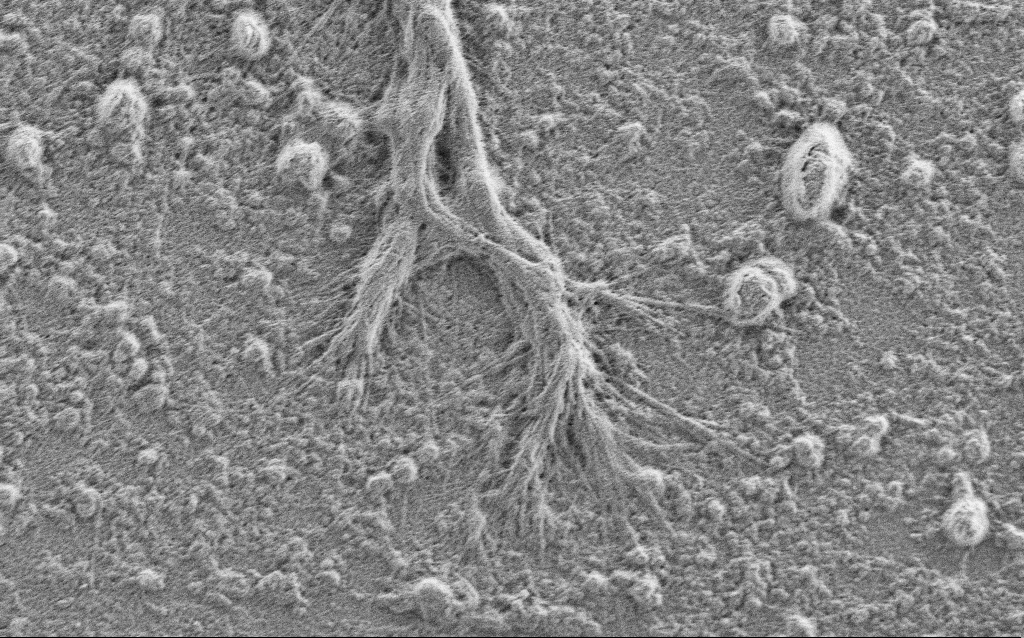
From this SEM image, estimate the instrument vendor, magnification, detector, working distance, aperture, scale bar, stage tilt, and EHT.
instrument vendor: Zeiss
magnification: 7.5 K X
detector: SE2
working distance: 4 mm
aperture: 30 µm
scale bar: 2000 nm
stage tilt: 0°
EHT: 0.9 kV